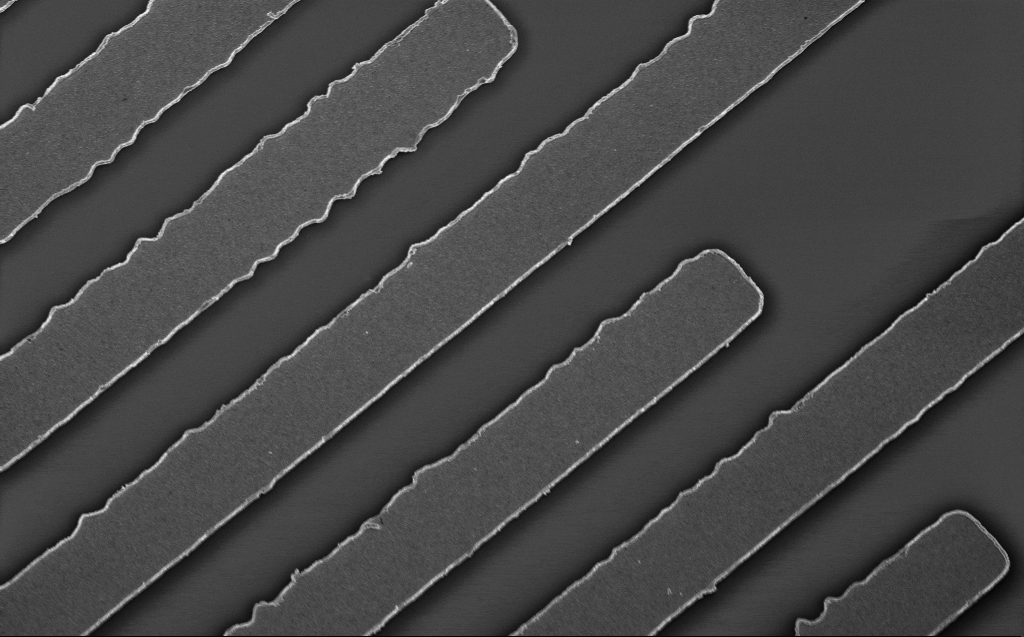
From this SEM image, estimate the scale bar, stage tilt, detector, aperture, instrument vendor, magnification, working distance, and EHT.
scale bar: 2000 nm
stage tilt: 20°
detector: InLens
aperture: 30 µm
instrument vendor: Zeiss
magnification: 11.6 K X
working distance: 4 mm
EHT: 3 kV